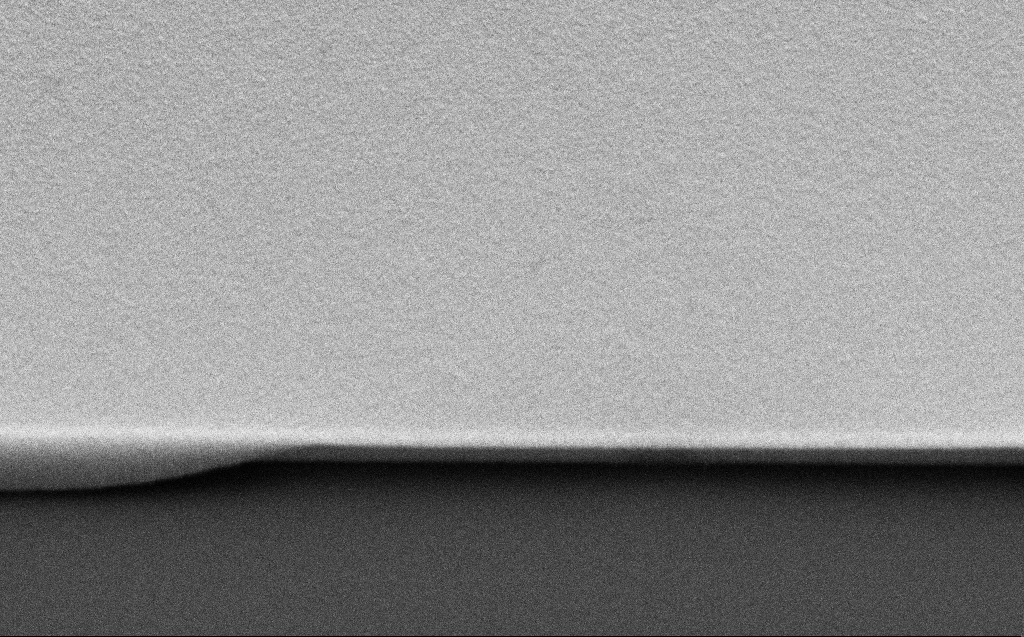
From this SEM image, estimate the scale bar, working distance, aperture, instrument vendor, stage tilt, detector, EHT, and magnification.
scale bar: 2000 nm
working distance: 5 mm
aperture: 30 µm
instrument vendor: Zeiss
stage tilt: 30°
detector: SE2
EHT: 1.1 kV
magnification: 14.61 K X